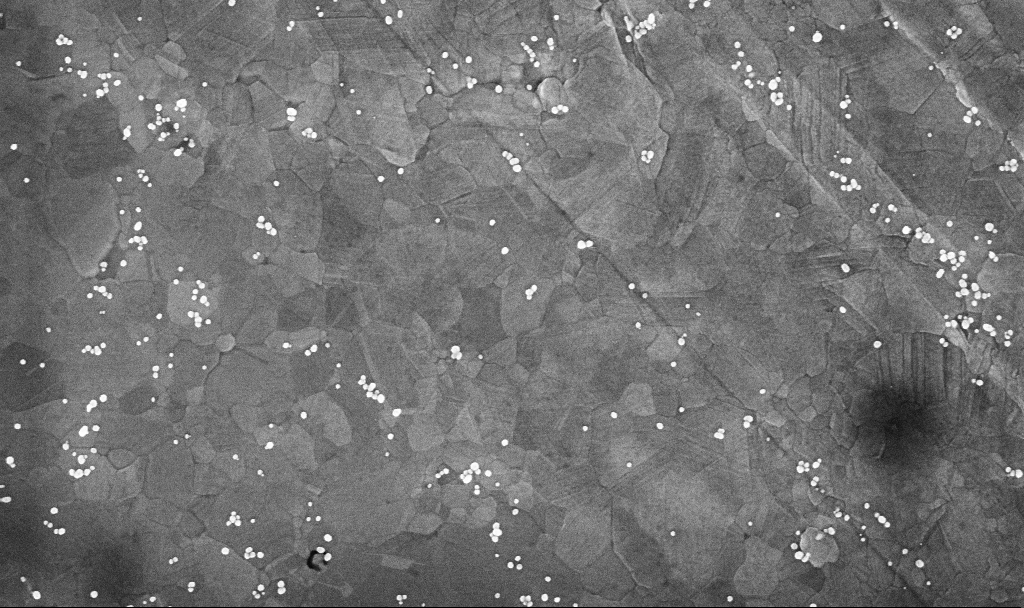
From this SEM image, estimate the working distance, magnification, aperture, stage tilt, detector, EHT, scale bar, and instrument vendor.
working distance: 3.4 mm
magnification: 100.42 K X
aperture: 30 µm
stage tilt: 0°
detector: InLens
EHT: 10 kV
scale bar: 200 nm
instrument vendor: Zeiss